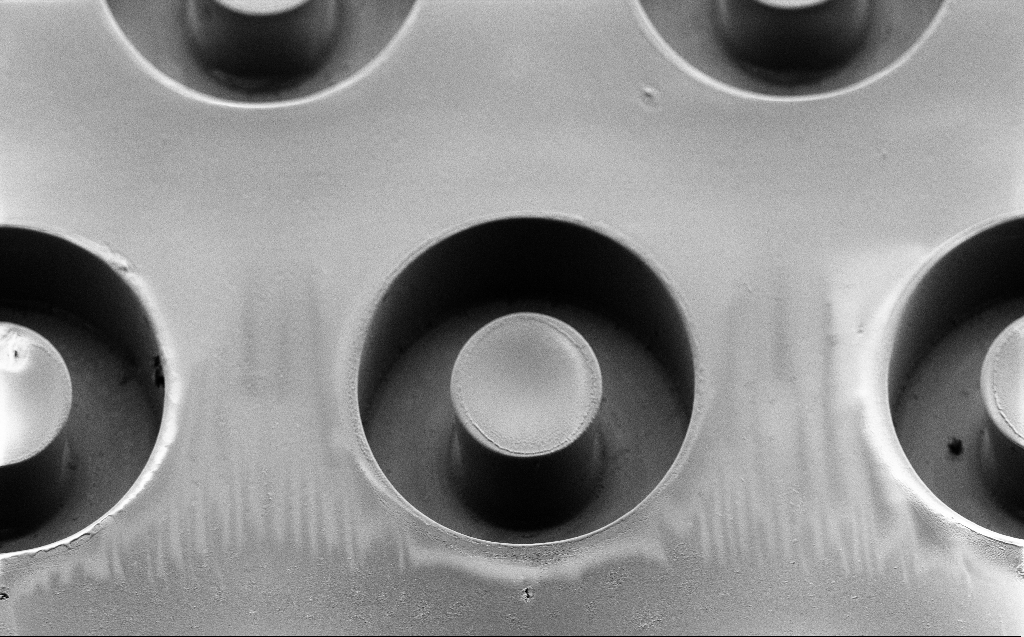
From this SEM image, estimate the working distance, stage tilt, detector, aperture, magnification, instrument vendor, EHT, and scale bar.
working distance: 6 mm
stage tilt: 45°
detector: SE2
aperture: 30 µm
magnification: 1.4 K X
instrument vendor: Zeiss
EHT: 2 kV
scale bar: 20000 nm